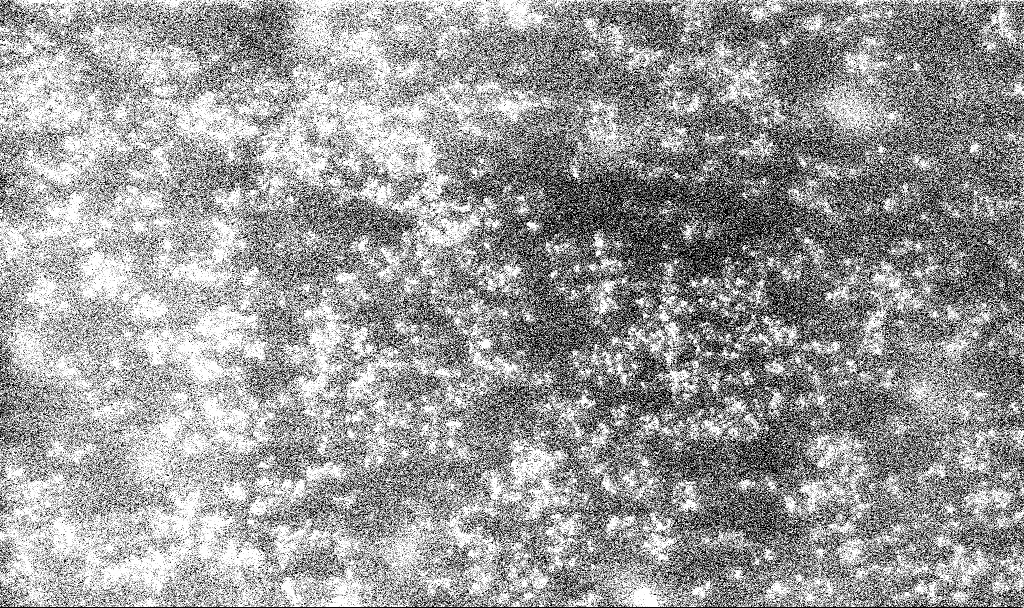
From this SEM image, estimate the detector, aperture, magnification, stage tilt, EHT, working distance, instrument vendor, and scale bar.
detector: InLens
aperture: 30 µm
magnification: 8.63 K X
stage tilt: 0°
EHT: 10 kV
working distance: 2.4 mm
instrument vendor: Zeiss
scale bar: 2000 nm